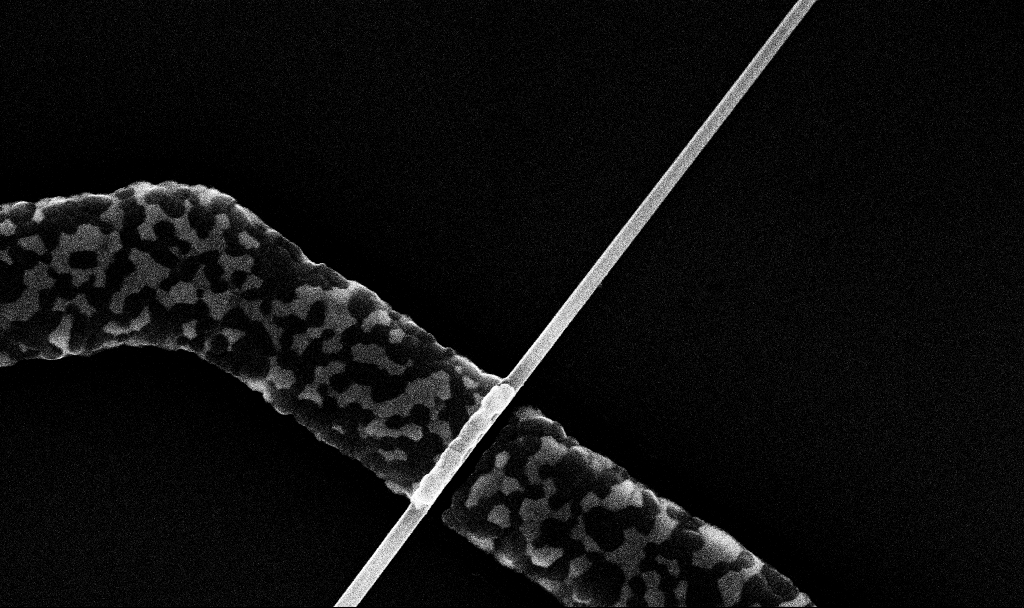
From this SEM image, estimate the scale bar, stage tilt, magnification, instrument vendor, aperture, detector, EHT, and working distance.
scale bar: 1000 nm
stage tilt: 0°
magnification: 63.86 K X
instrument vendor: Zeiss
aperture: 30 µm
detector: InLens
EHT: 10 kV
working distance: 6.7 mm